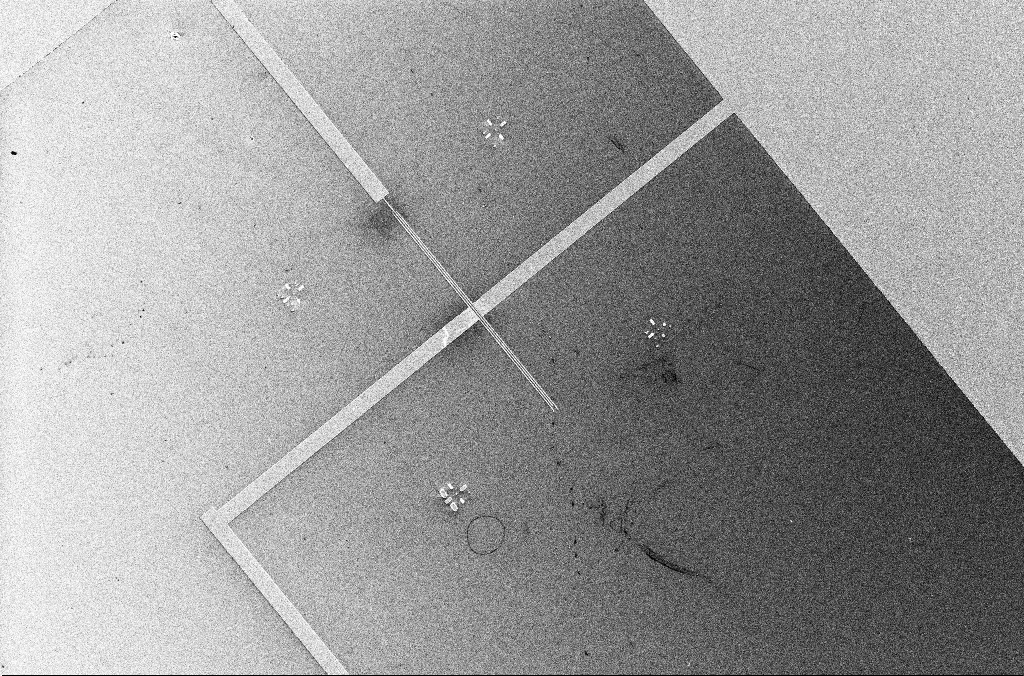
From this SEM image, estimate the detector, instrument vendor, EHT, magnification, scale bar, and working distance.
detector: InLens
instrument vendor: Zeiss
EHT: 5 kV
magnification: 1.2 K X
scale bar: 20000 nm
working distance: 3.4 mm